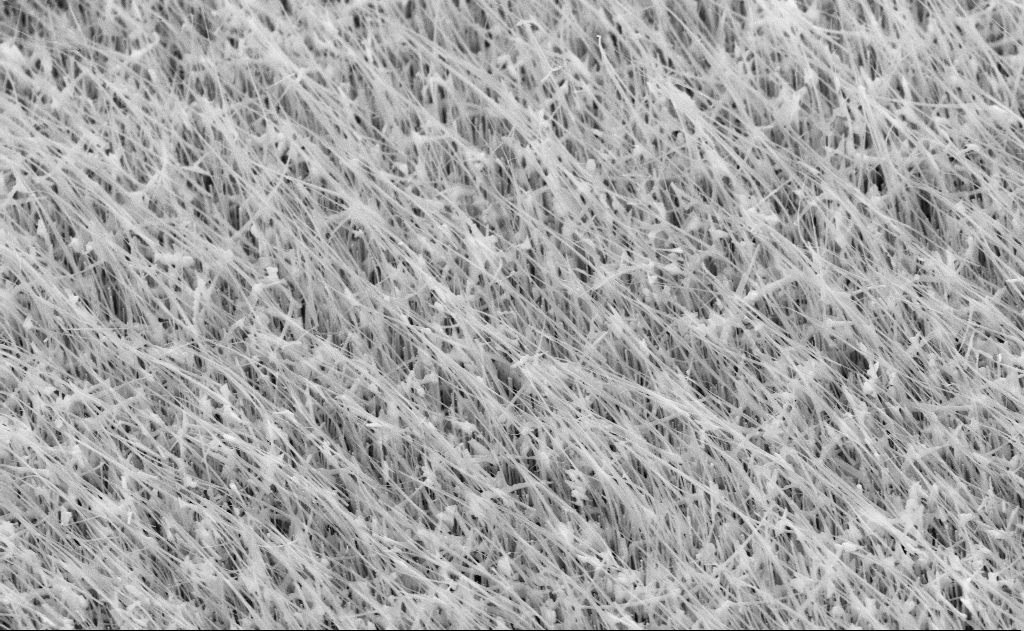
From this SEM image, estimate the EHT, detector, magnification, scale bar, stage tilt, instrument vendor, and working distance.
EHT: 10 kV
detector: SE2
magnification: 20 K X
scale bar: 1000 nm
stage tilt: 45°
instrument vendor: Zeiss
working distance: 12 mm